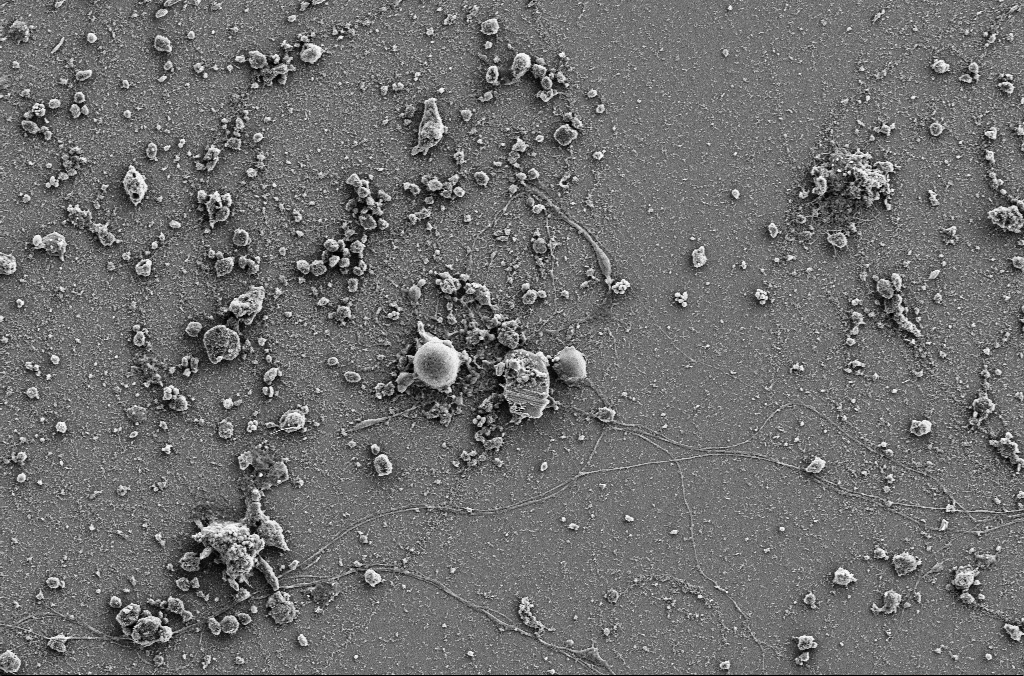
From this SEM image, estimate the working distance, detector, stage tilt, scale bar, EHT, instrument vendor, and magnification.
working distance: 4 mm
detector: SE2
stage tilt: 0°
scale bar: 10000 nm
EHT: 5 kV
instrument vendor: Zeiss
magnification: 2 K X